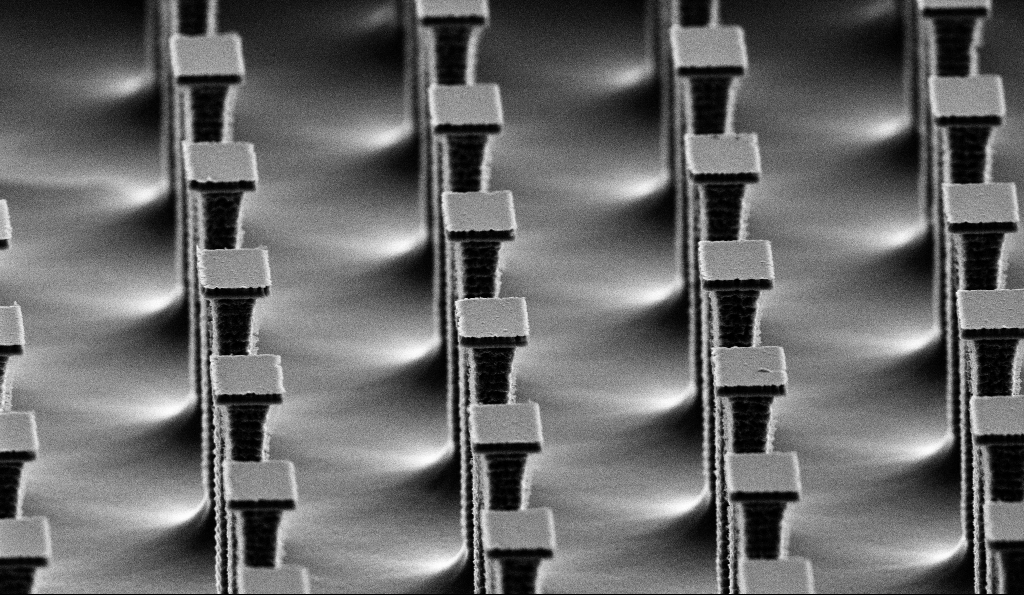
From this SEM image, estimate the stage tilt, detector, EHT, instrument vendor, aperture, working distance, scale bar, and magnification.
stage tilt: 70°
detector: SE2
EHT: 5 kV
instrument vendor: Zeiss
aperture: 30 µm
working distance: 10.6 mm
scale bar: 2000 nm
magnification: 8.79 K X